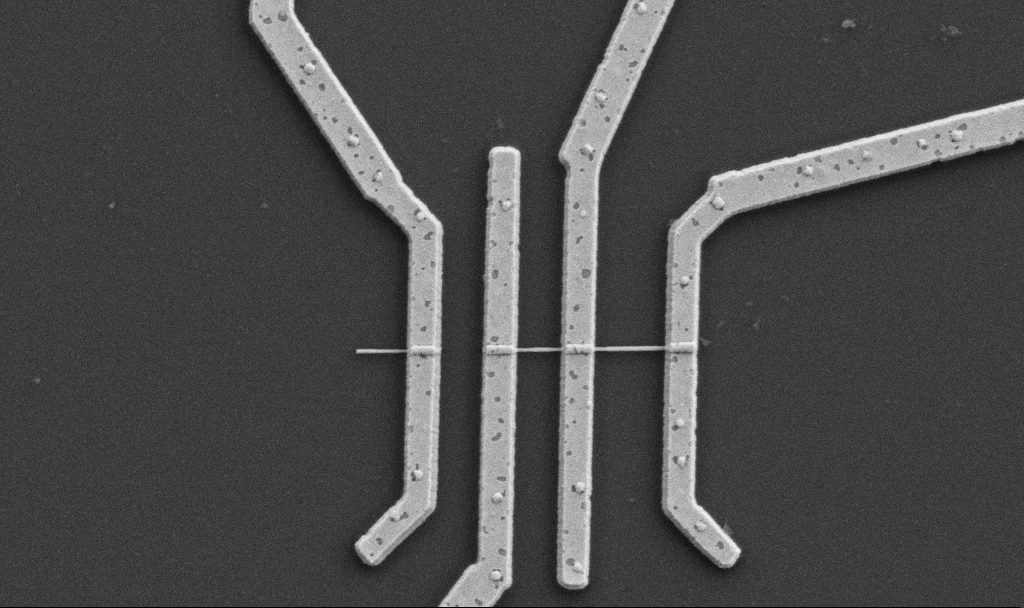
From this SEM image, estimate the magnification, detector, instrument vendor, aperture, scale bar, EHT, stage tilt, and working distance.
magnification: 20 K X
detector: SE2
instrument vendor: Zeiss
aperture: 30 µm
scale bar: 1000 nm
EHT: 5 kV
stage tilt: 0°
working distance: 10.6 mm